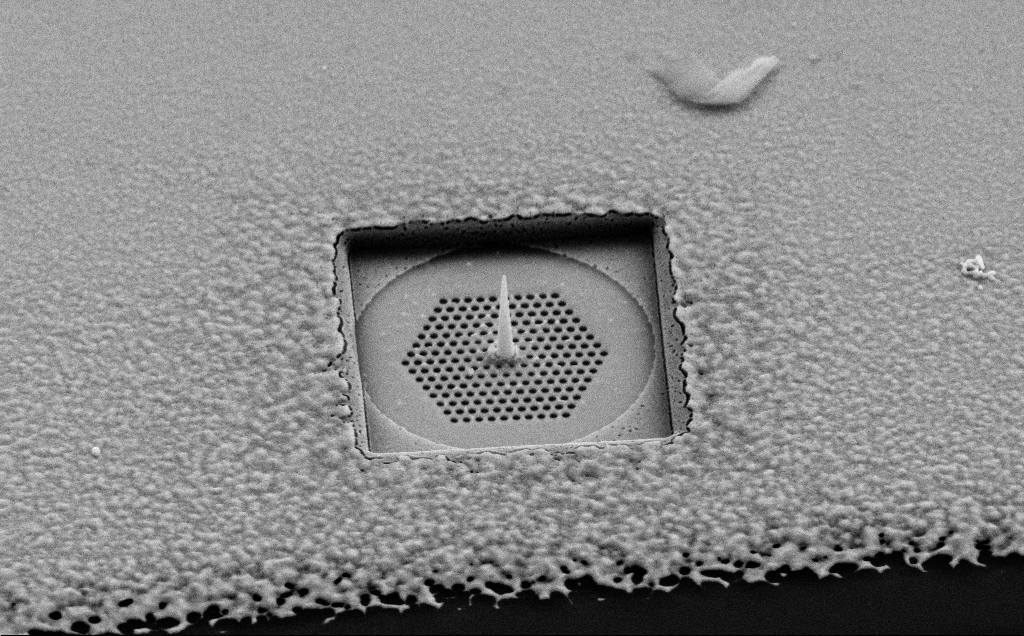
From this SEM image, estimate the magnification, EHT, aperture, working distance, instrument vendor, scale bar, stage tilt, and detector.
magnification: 18.34 K X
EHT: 10 kV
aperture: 30 µm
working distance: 8 mm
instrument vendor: Zeiss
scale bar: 2000 nm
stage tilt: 45°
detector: SE2